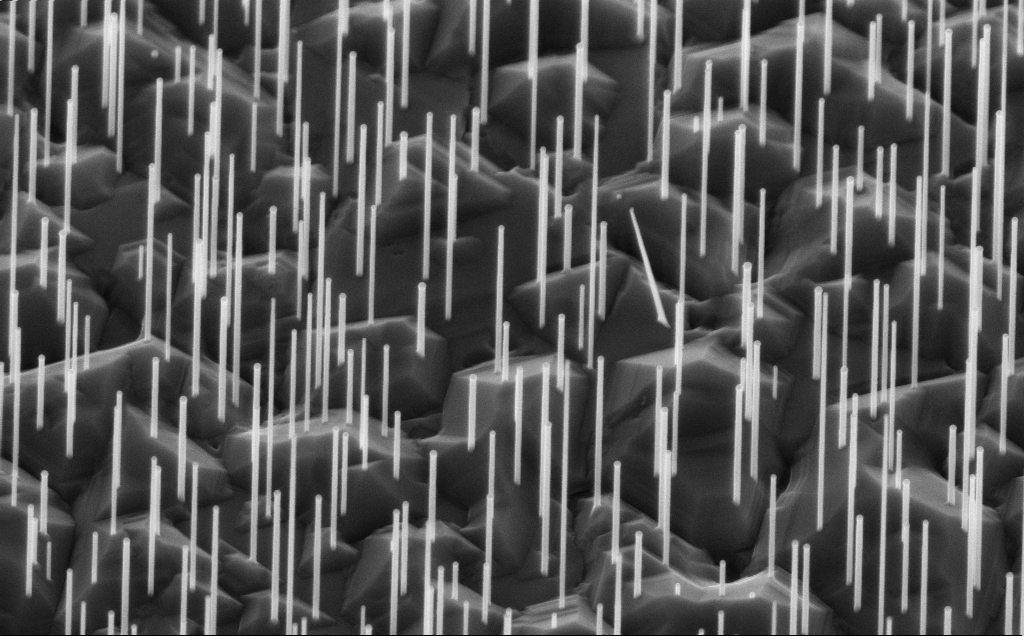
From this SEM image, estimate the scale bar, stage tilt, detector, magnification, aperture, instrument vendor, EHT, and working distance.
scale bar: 1000 nm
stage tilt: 45°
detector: InLens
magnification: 40 K X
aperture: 30 µm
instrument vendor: Zeiss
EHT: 10 kV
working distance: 6 mm